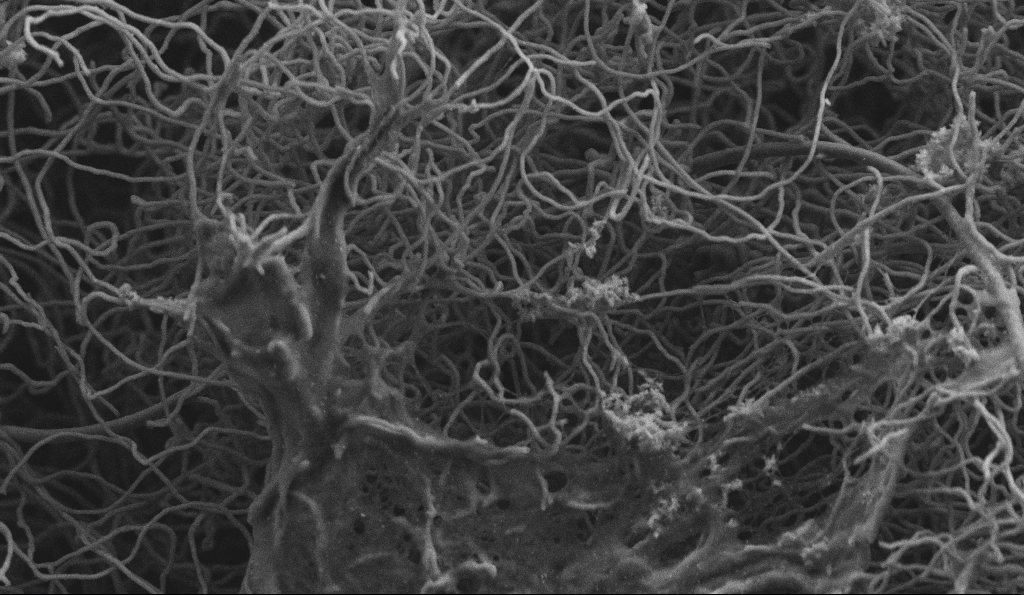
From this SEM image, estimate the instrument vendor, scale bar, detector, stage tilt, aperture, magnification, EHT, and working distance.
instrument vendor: Zeiss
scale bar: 1000 nm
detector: SE2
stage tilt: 0°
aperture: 30 µm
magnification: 15 K X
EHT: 3 kV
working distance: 4.6 mm